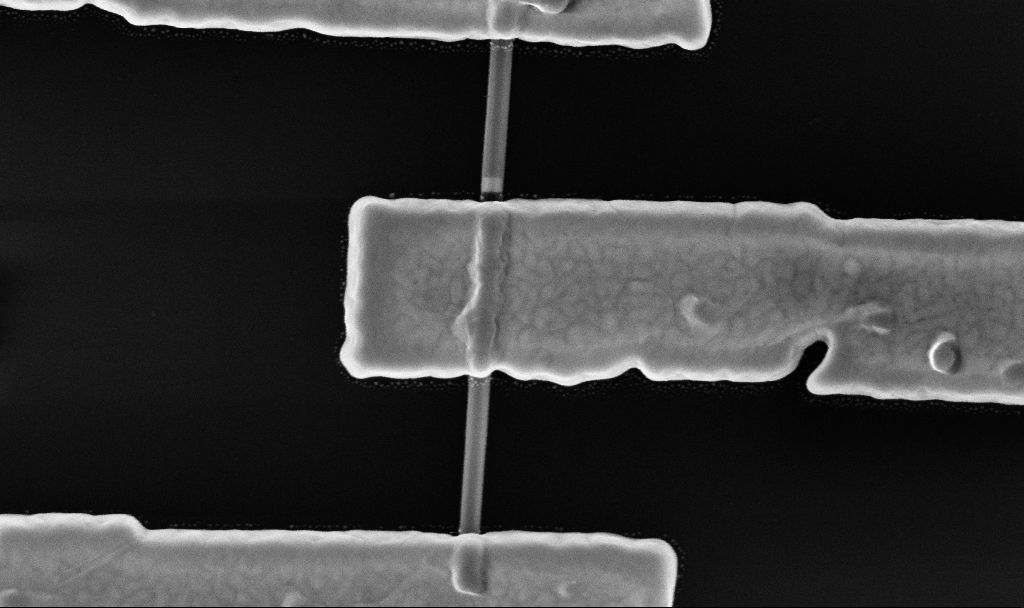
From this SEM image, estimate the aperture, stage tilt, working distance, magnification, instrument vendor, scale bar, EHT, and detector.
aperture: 30 µm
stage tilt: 0°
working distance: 6.7 mm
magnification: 128.09 K X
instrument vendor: Zeiss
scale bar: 200 nm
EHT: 10 kV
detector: InLens